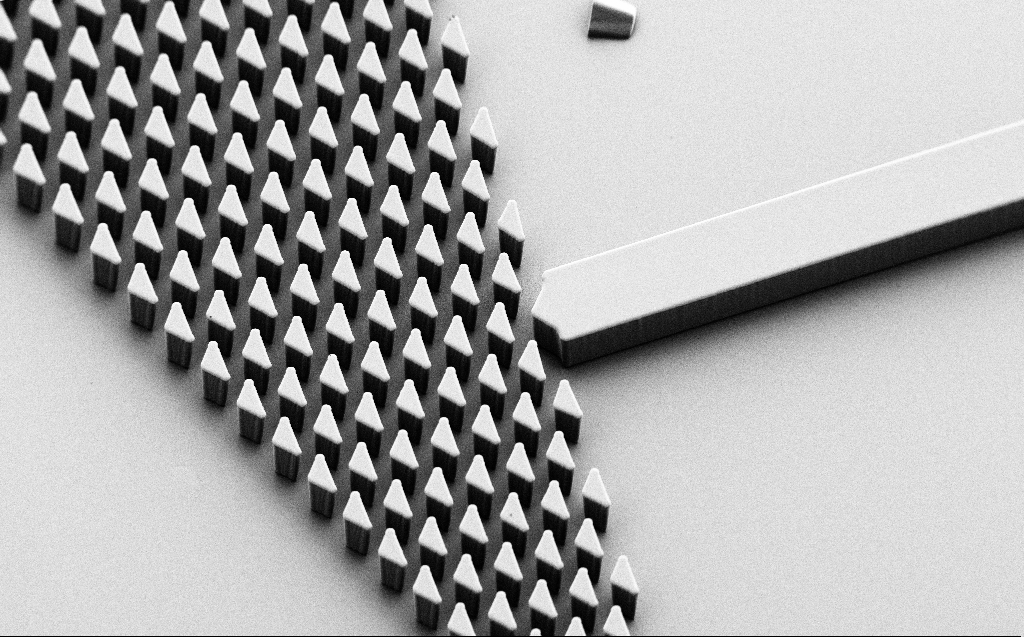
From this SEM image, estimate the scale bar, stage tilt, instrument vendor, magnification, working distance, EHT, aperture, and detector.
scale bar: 100000 nm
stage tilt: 45°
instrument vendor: Zeiss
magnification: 0.453 K X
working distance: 6 mm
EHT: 1 kV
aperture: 30 µm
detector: SE2